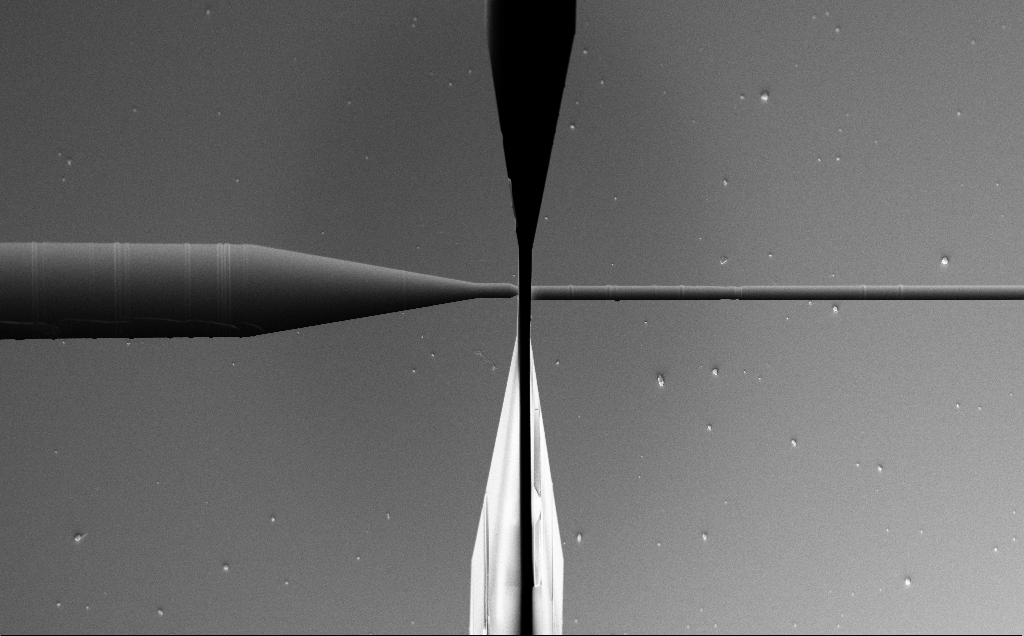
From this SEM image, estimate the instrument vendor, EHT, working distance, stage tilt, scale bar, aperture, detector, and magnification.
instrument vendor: Zeiss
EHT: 5 kV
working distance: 7 mm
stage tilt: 45°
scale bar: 100000 nm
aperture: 30 µm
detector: InLens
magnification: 0.415 K X